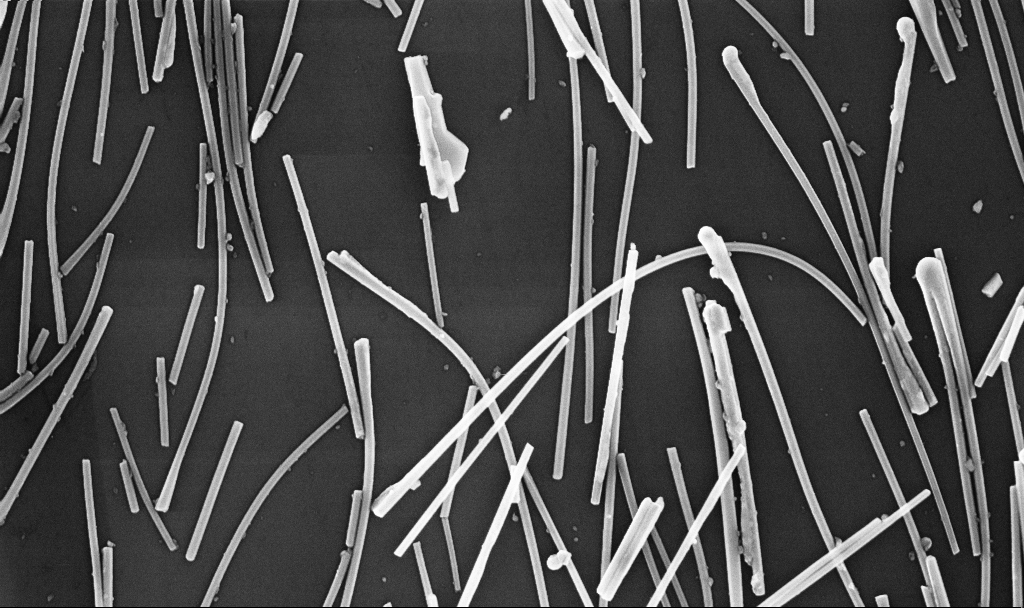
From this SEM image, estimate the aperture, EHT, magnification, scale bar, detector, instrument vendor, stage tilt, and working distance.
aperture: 30 µm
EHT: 10 kV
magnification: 29.32 K X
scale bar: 1000 nm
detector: InLens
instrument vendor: Zeiss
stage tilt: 0°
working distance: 6.7 mm